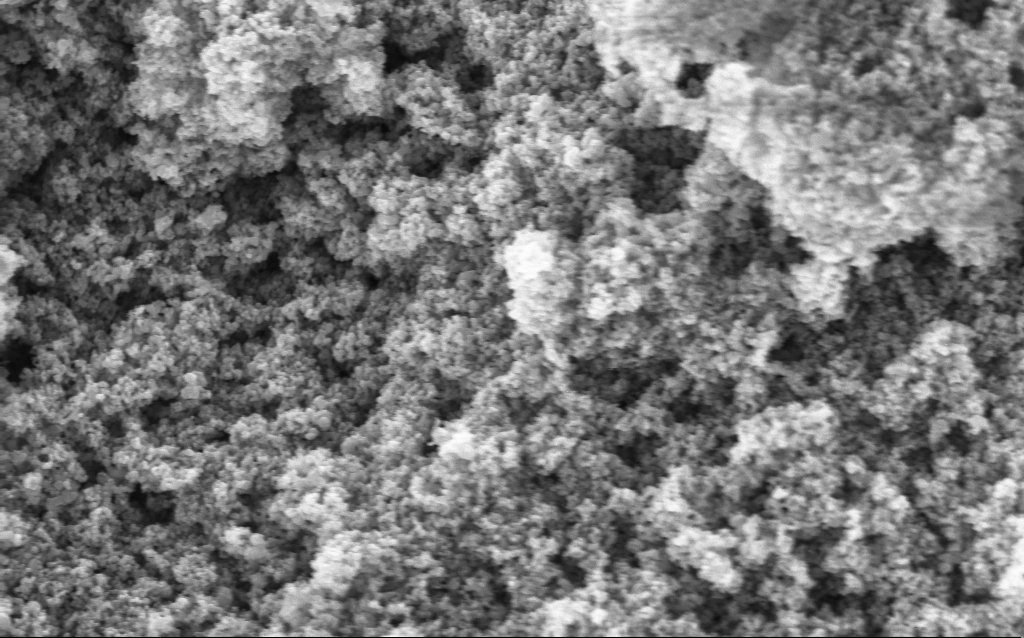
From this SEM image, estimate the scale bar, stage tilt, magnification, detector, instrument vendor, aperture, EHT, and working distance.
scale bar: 1000 nm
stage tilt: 0°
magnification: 68.69 K X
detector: InLens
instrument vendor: Zeiss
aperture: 30 µm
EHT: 5 kV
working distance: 4.4 mm